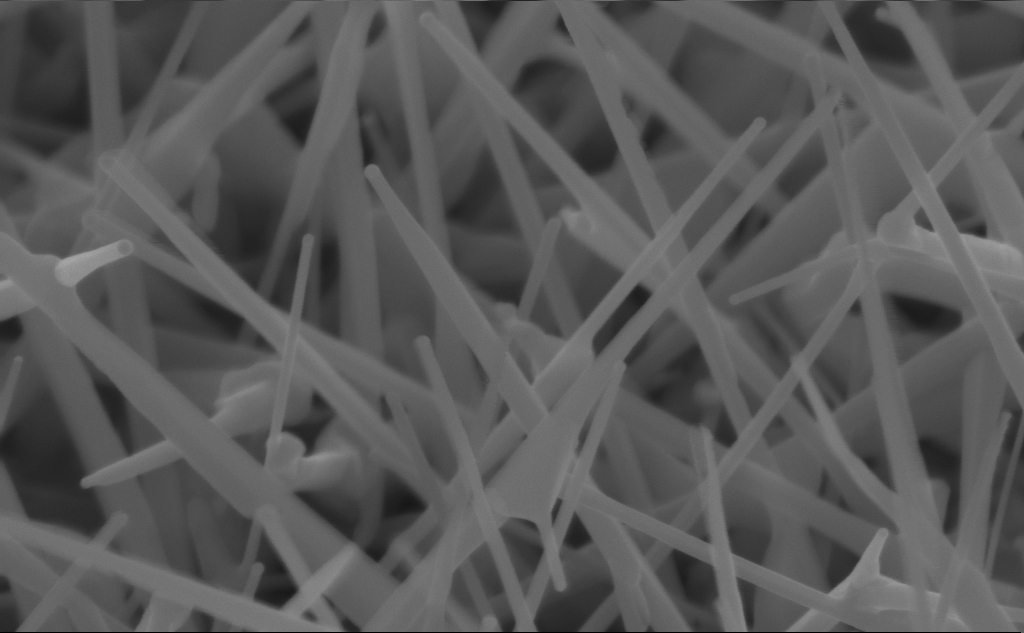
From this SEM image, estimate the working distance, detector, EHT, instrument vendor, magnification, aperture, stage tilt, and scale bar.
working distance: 4 mm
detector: InLens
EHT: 10 kV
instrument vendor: Zeiss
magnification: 150 K X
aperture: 30 µm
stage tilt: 45°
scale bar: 200 nm